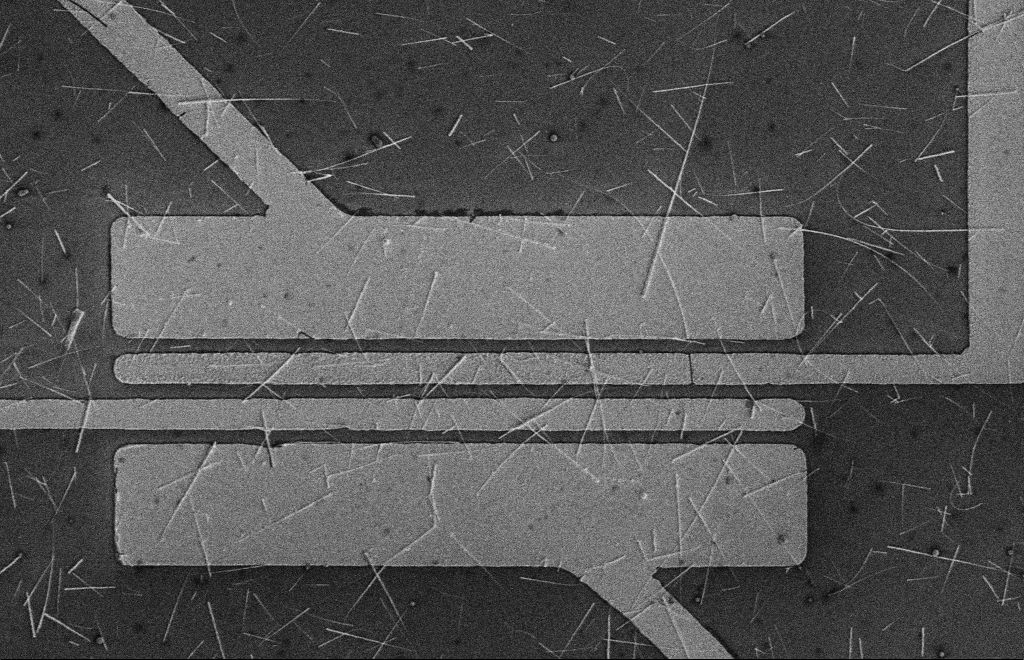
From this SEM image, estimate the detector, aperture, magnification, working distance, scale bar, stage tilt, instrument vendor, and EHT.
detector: SE2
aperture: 10 µm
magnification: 4.2 K X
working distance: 16 mm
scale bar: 10000 nm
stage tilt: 0°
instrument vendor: Zeiss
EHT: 5 kV